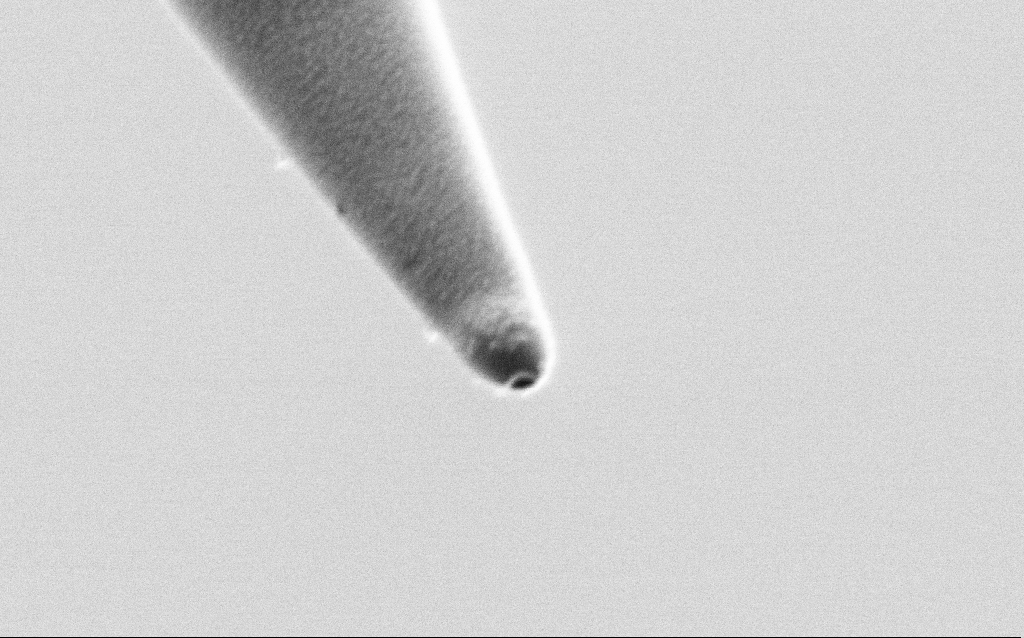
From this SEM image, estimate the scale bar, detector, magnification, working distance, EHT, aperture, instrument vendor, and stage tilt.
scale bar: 200 nm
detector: SE2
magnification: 100 K X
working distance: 7.2 mm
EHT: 1 kV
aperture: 30 µm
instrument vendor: Zeiss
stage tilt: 45°